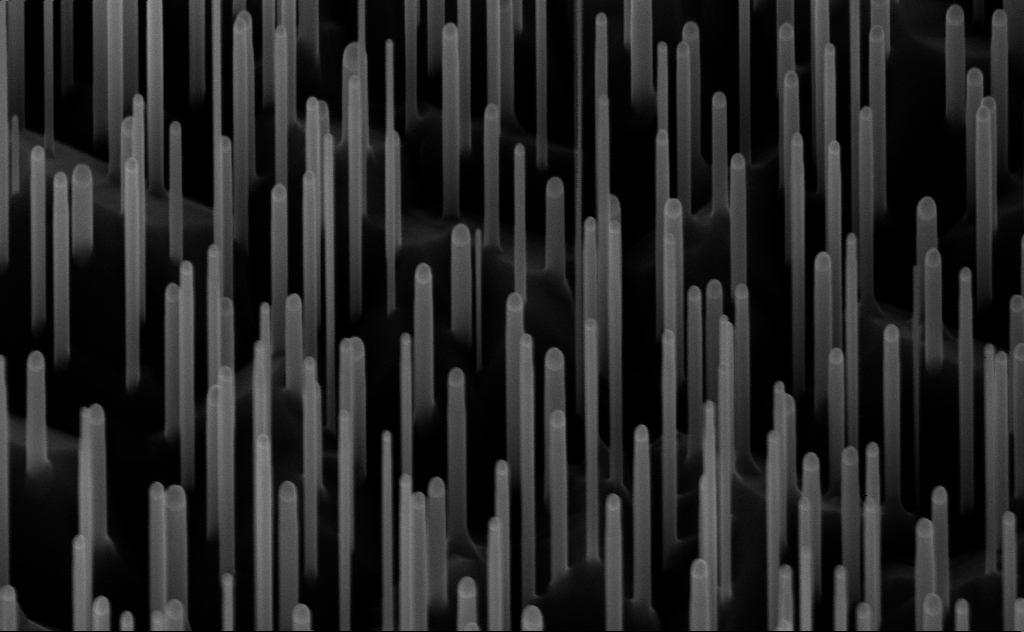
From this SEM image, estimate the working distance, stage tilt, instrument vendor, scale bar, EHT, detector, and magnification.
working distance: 7 mm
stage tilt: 45°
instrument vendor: Zeiss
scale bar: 200 nm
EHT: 10 kV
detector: InLens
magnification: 80 K X